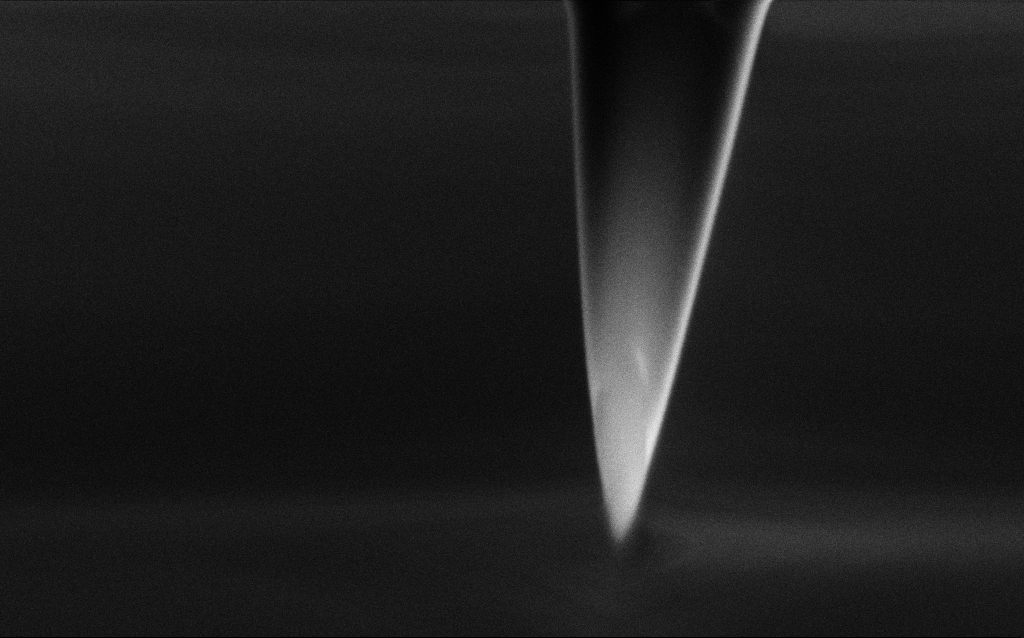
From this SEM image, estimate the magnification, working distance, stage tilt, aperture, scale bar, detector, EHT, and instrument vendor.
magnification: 77.37 K X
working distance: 5 mm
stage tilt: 45°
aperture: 30 µm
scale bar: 200 nm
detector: SE2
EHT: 2 kV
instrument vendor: Zeiss